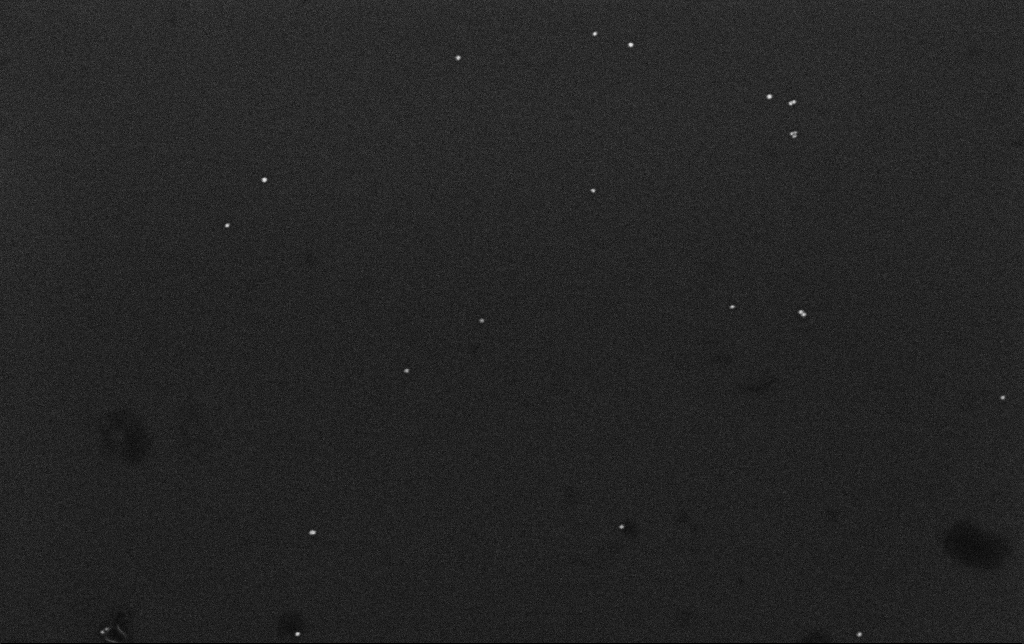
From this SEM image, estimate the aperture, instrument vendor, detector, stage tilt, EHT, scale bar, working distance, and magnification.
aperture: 30 µm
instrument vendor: Zeiss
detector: InLens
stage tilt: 0°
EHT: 10 kV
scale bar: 200 nm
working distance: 3.2 mm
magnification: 100 K X